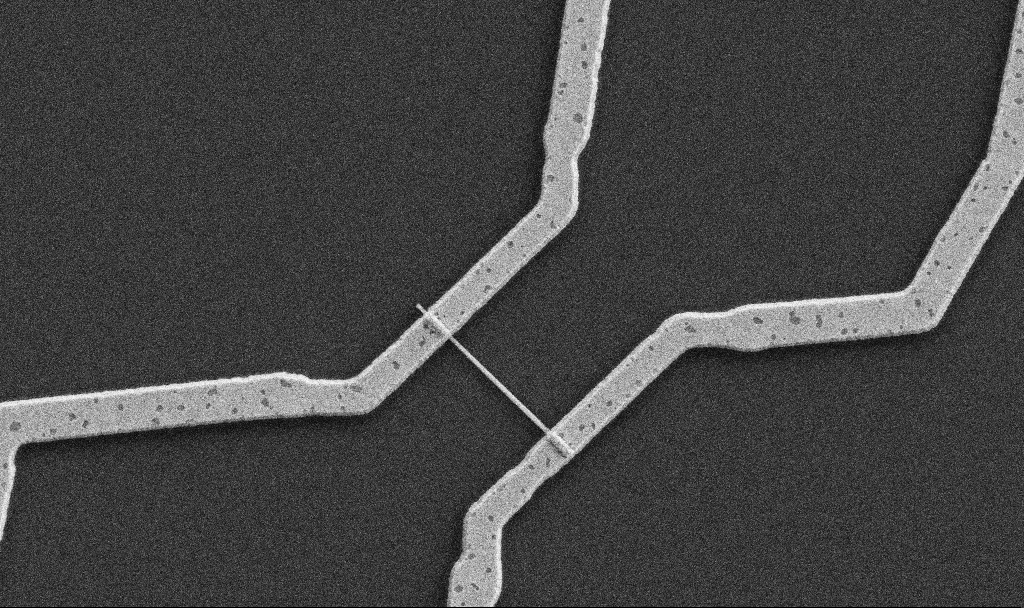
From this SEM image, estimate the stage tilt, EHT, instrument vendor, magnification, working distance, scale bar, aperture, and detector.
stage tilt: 0°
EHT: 5 kV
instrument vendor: Zeiss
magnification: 20 K X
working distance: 10.7 mm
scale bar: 1000 nm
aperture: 30 µm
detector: SE2